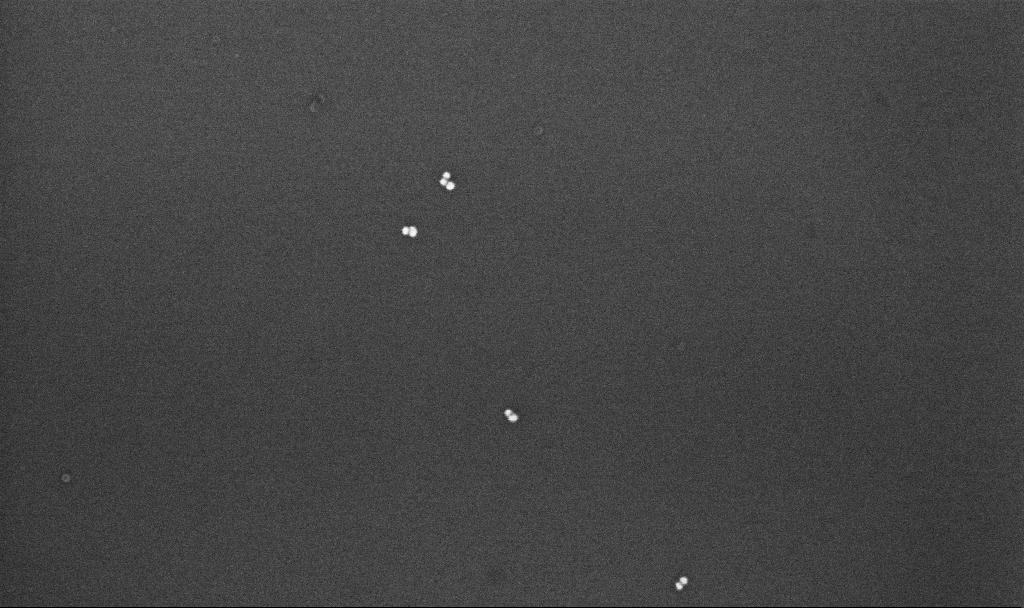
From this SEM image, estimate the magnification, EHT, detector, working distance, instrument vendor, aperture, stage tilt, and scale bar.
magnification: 167.02 K X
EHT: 10 kV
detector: InLens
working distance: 4.3 mm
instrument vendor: Zeiss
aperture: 30 µm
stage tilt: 0°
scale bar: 200 nm